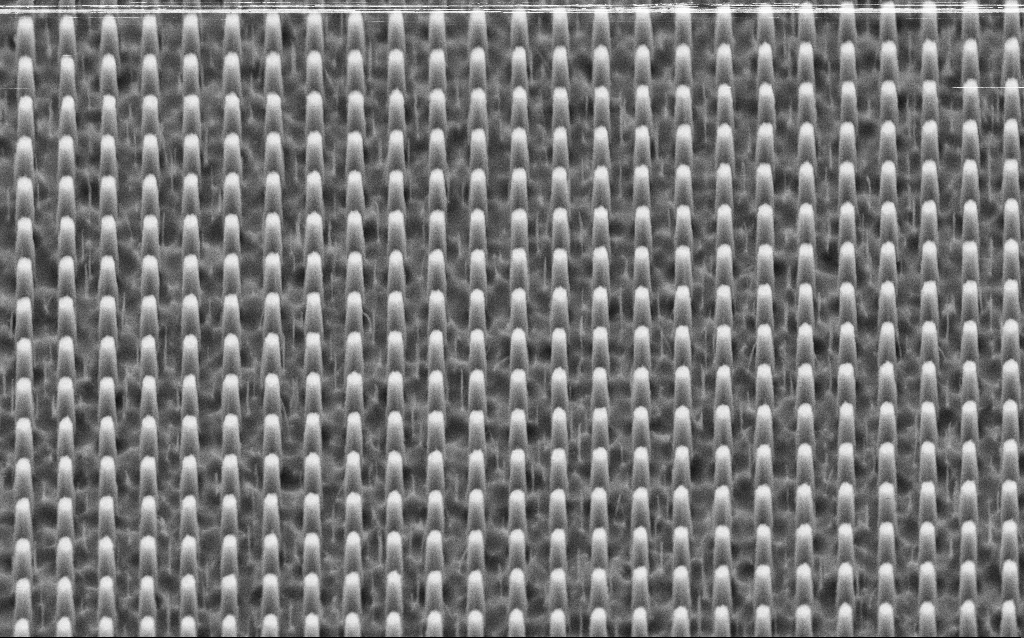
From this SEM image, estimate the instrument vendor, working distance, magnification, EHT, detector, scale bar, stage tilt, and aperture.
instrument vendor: Zeiss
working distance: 5 mm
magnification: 38.15 K X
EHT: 3 kV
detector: SE2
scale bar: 1000 nm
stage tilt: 45°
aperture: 30 µm